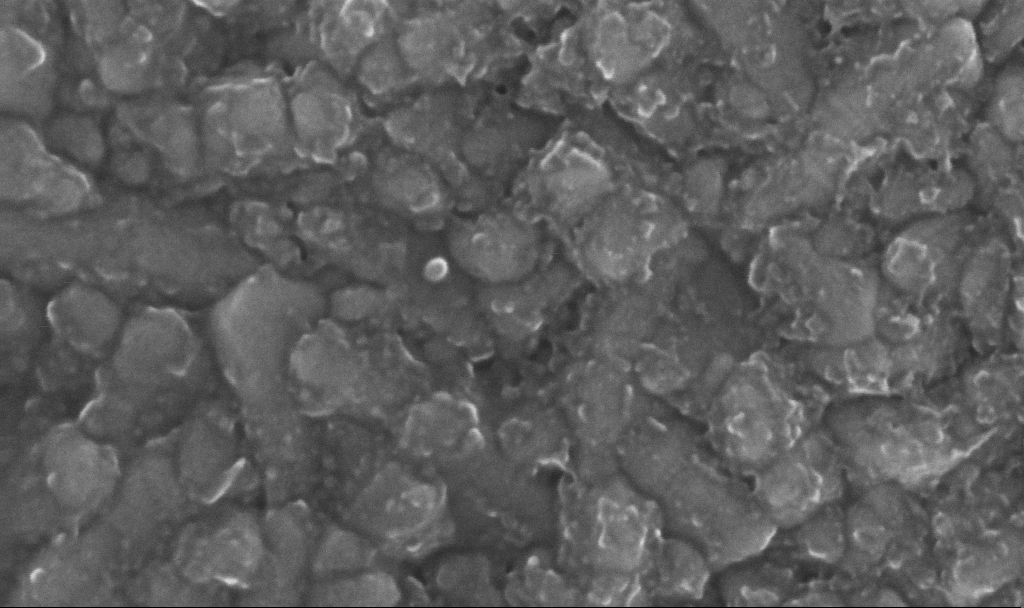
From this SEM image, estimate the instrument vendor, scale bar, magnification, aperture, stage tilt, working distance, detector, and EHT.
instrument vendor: Zeiss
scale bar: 100 nm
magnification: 400.61 K X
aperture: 30 µm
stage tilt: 0°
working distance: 4.8 mm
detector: InLens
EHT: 20 kV